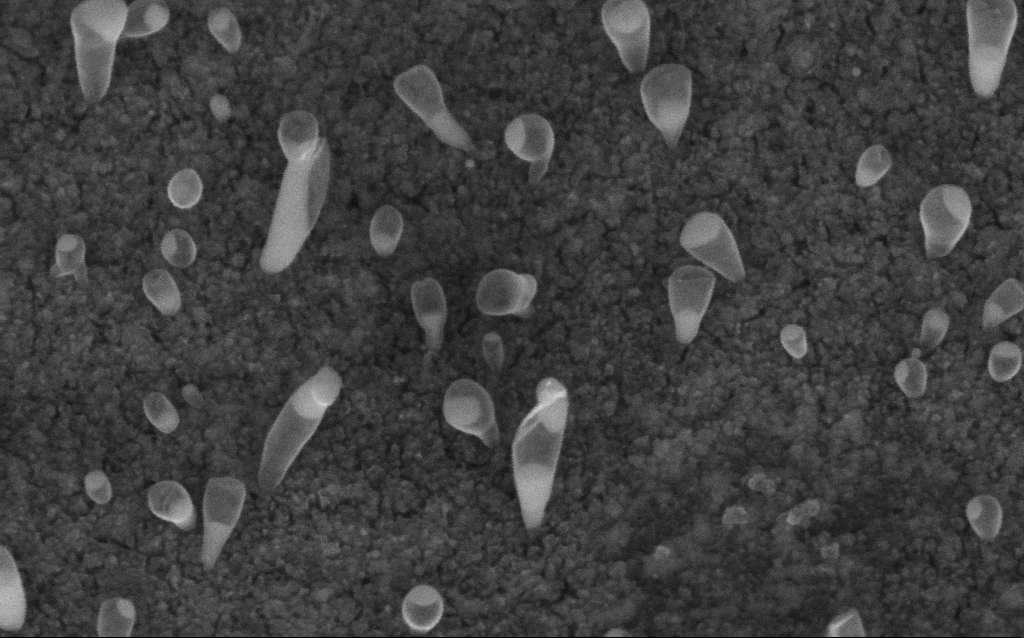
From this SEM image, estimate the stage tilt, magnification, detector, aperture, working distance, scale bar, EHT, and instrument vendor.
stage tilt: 45°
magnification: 200 K X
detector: InLens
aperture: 30 µm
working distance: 6.7 mm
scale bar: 100 nm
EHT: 5 kV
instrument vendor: Zeiss